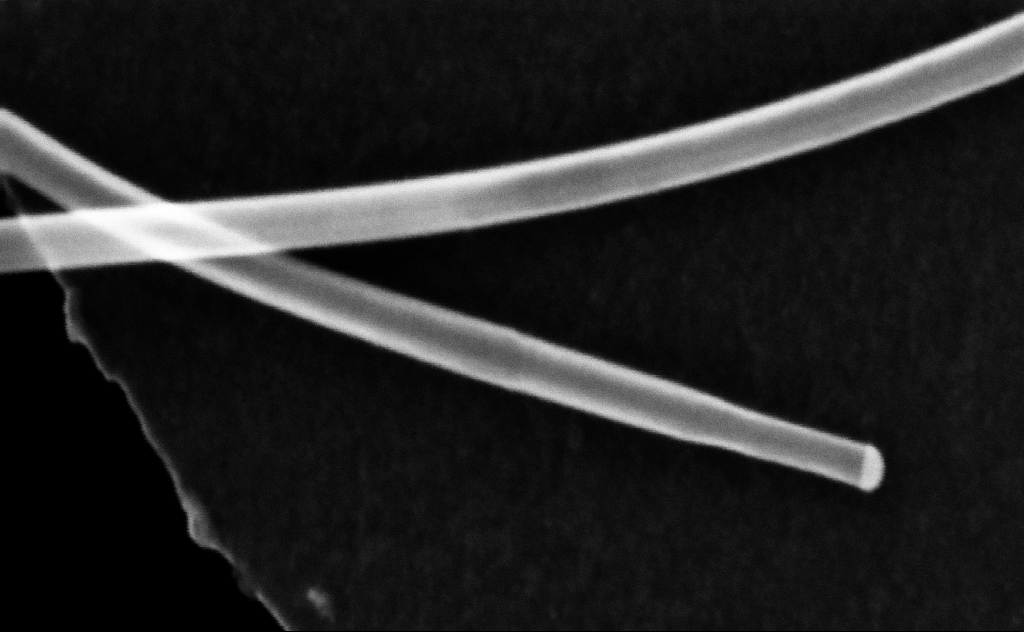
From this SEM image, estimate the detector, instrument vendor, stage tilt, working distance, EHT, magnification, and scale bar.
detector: InLens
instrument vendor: Zeiss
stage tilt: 0°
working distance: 8 mm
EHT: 20 kV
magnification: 487.23 K X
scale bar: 100 nm